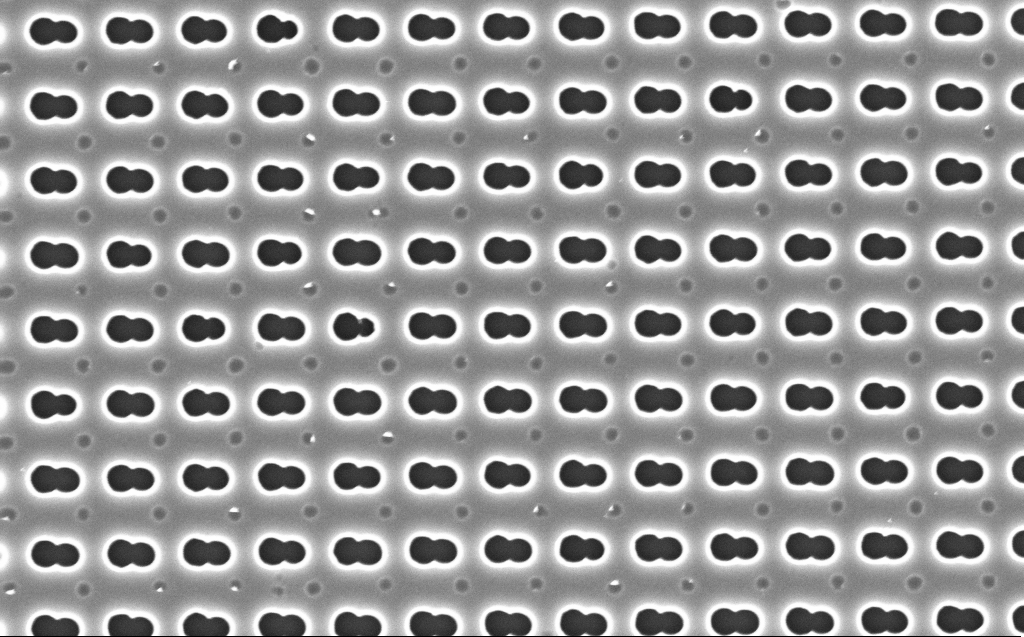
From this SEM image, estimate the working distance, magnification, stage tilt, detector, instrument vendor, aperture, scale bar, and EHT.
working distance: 4 mm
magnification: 26.48 K X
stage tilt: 0°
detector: InLens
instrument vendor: Zeiss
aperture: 30 µm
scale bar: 2000 nm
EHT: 5 kV